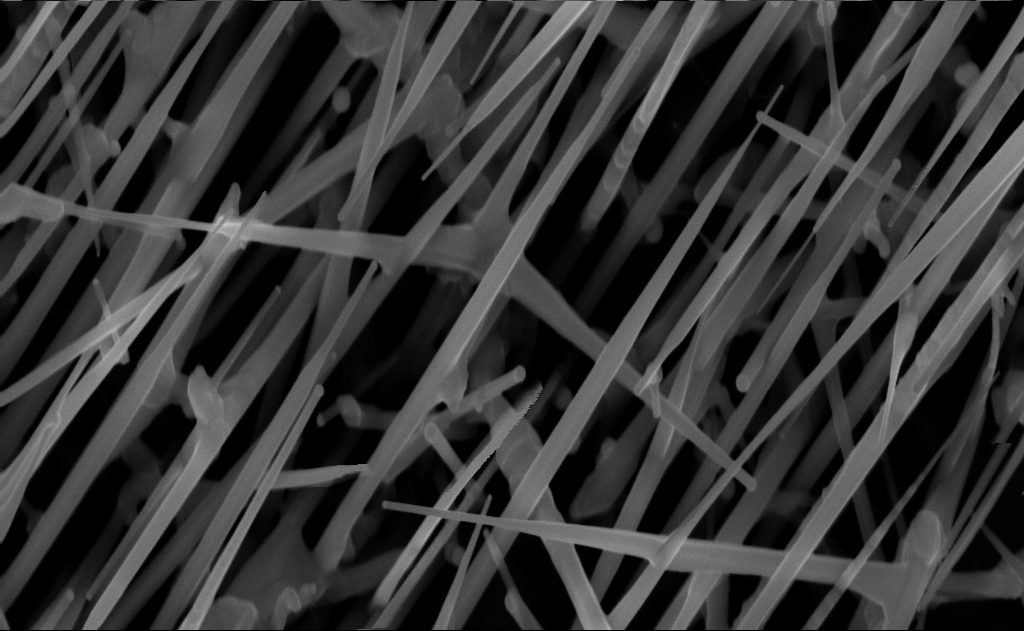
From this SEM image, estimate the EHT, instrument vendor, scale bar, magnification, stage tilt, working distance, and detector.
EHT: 10 kV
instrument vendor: Zeiss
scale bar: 200 nm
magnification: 80 K X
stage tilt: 0°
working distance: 7 mm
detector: InLens